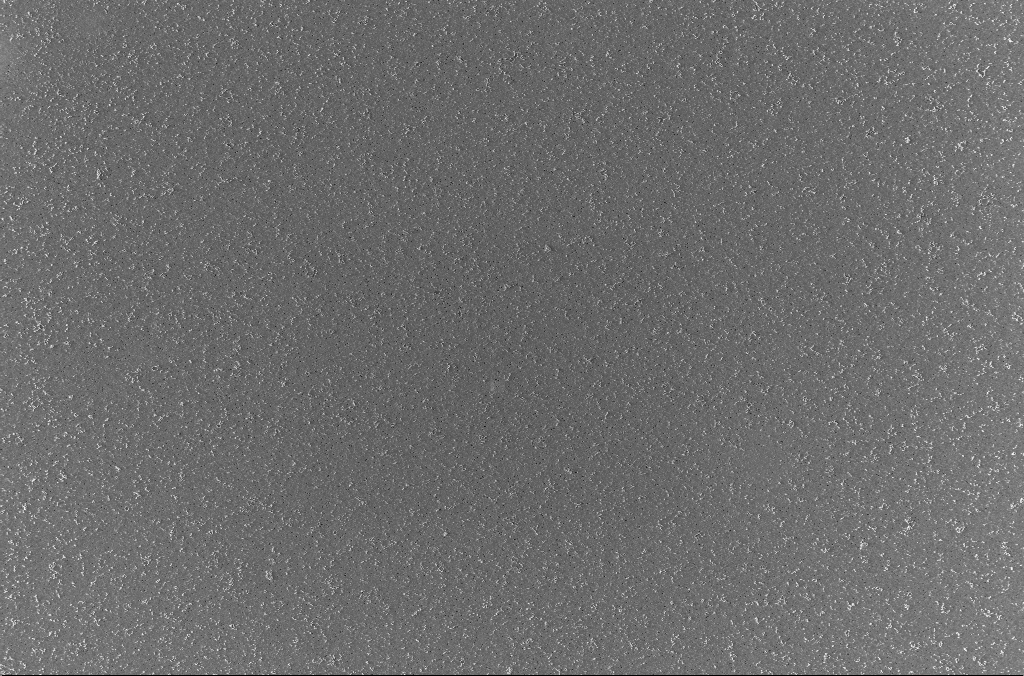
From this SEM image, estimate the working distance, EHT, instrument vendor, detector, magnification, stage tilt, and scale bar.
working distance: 3 mm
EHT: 2 kV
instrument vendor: Zeiss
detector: InLens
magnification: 1 K X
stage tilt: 0°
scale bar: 20000 nm